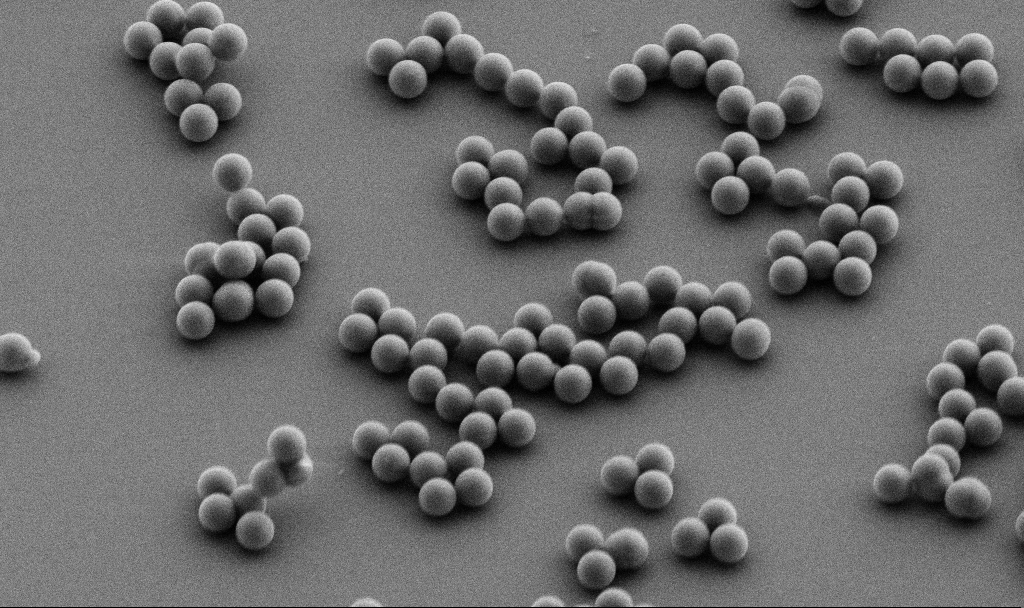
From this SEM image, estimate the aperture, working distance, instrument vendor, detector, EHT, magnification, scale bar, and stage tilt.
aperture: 30 µm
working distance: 8 mm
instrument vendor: Zeiss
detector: SE2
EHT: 5 kV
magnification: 11.08 K X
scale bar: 2000 nm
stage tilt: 45°